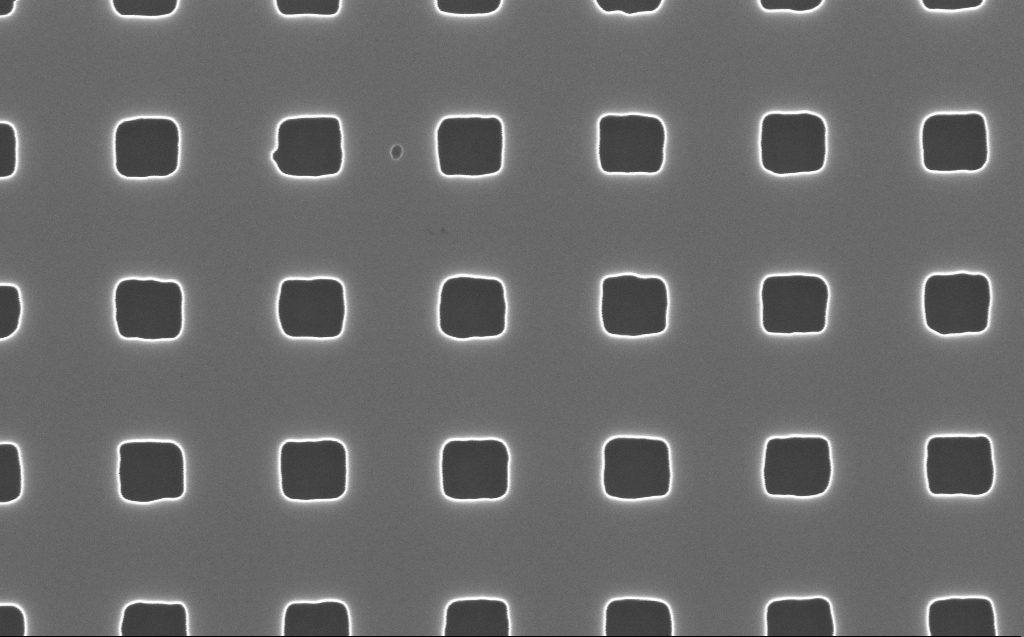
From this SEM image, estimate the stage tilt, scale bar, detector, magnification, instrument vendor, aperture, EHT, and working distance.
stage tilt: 0°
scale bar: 100 nm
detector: InLens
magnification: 120 K X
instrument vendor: Zeiss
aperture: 30 µm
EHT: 10 kV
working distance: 6 mm